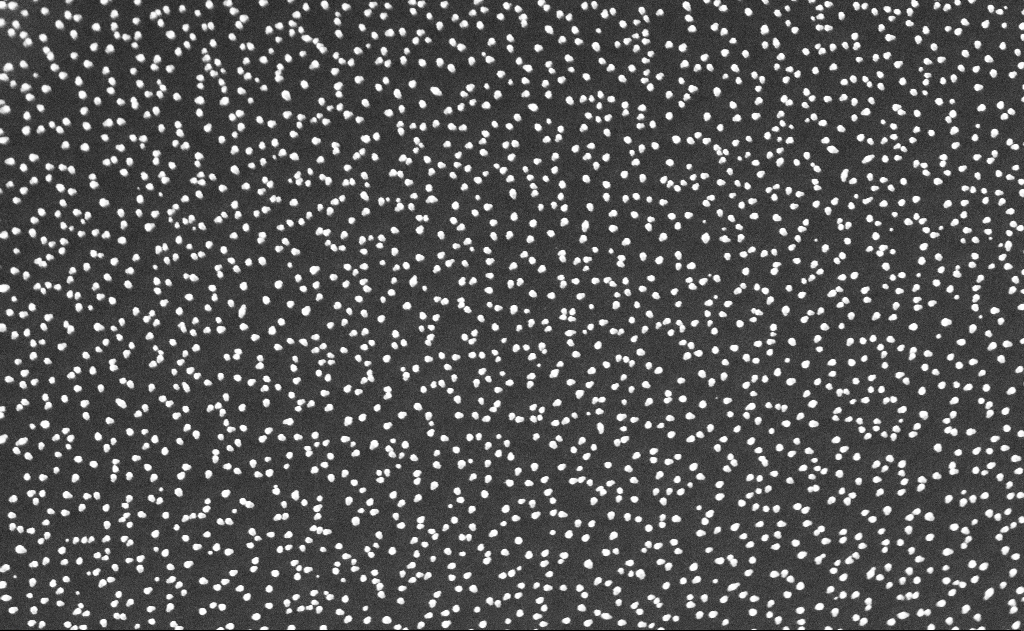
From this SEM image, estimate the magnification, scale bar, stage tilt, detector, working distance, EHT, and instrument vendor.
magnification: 50 K X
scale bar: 1000 nm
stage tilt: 0°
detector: InLens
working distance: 12 mm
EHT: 10 kV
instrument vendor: Zeiss